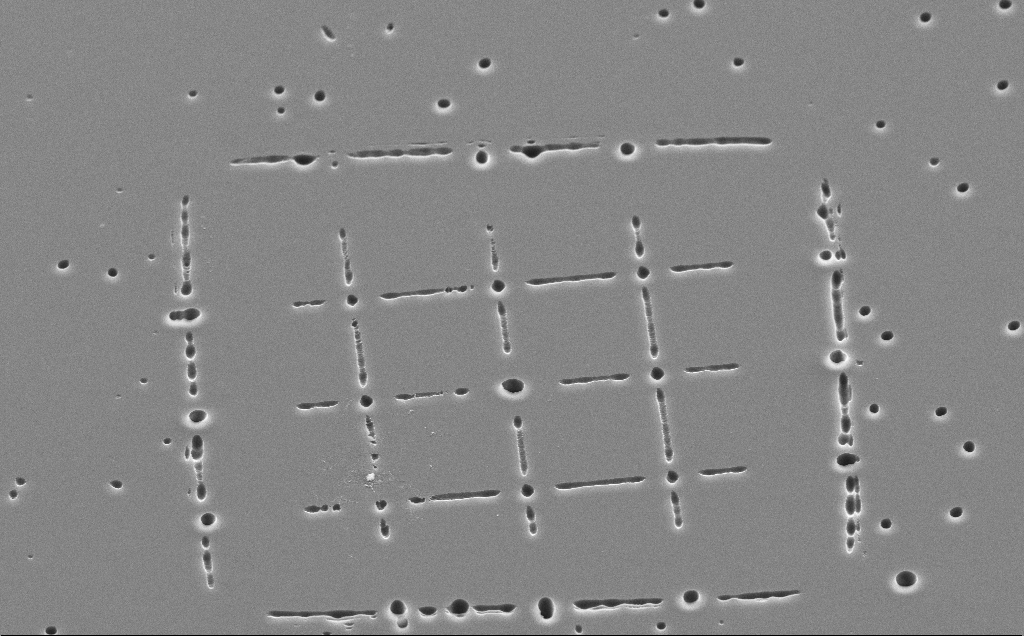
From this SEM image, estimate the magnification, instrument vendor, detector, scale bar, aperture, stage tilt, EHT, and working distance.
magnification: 2.64 K X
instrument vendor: Zeiss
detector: SE2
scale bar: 20000 nm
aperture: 30 µm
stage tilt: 45°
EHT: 10 kV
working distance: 12 mm